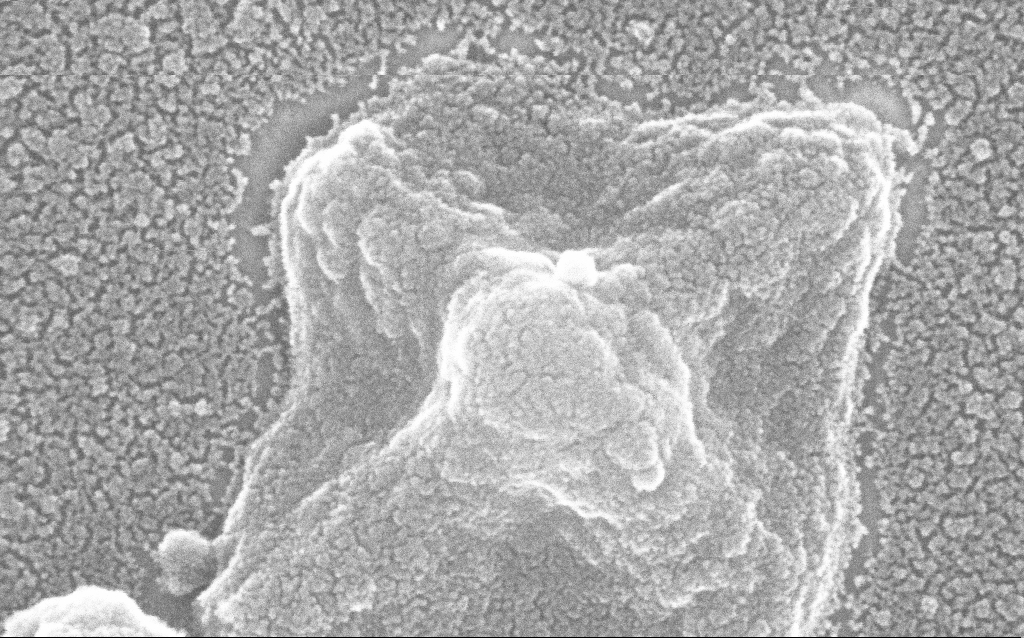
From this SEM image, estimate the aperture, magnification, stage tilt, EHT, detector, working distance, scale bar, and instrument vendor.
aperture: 30 µm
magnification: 500 K X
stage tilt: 0°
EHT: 20 kV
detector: InLens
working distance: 1.8 mm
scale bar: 100 nm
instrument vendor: Zeiss